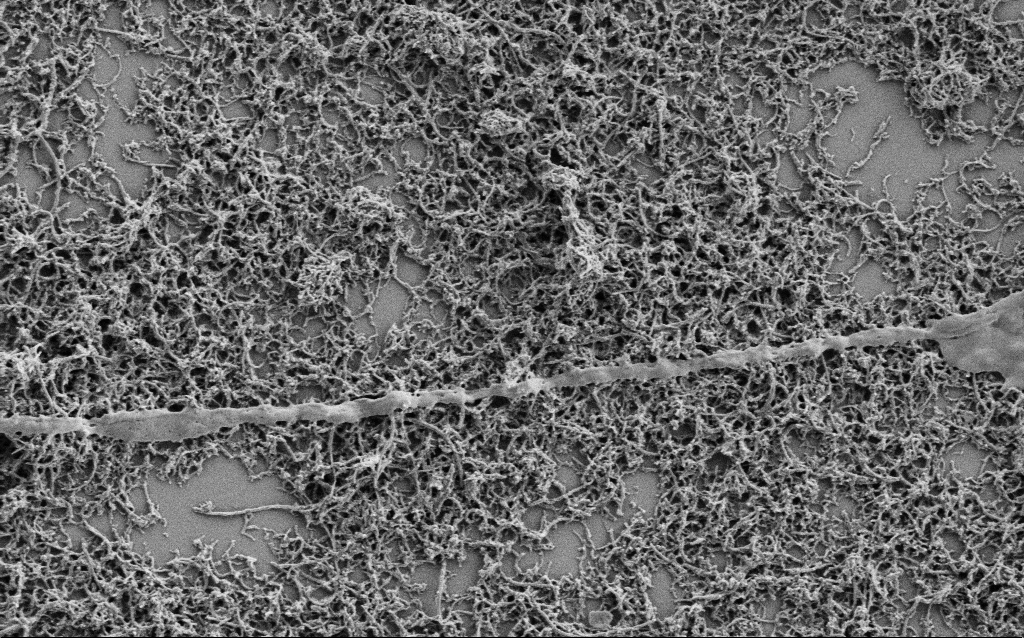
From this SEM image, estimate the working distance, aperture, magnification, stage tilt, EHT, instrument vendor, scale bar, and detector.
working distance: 7 mm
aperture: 30 µm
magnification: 25 K X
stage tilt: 0°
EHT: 1 kV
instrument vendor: Zeiss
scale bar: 1000 nm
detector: SE2